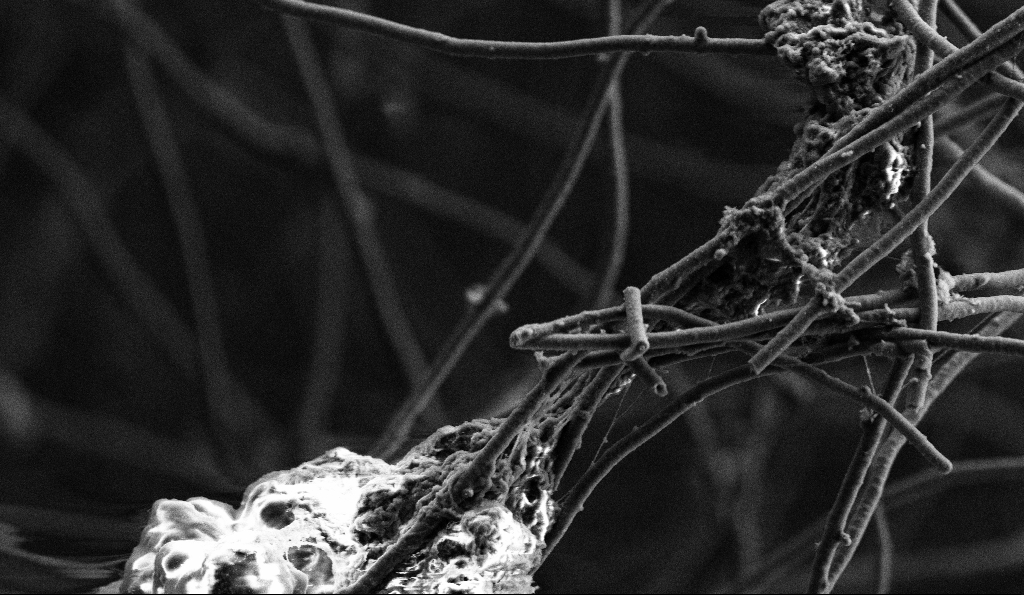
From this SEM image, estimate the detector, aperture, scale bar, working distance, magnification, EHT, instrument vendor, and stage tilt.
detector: SE2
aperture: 30 µm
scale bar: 2000 nm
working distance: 5.5 mm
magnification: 10 K X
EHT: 3 kV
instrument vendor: Zeiss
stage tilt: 0°